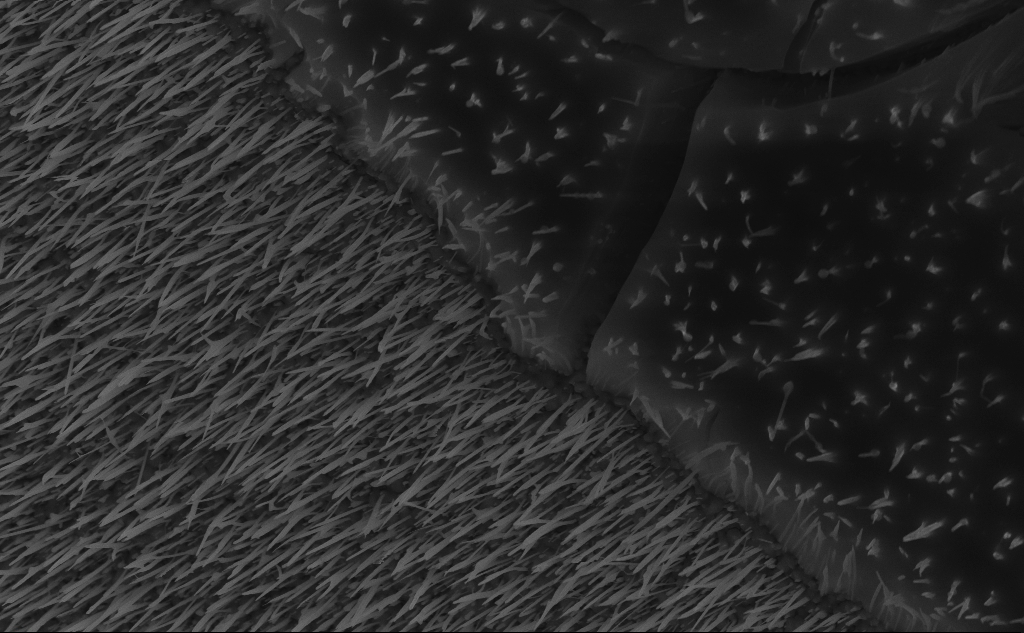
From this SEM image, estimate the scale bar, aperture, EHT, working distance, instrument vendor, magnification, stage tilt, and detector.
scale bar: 2000 nm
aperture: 30 µm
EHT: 10 kV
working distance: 6 mm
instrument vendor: Zeiss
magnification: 23.9 K X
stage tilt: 45°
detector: InLens